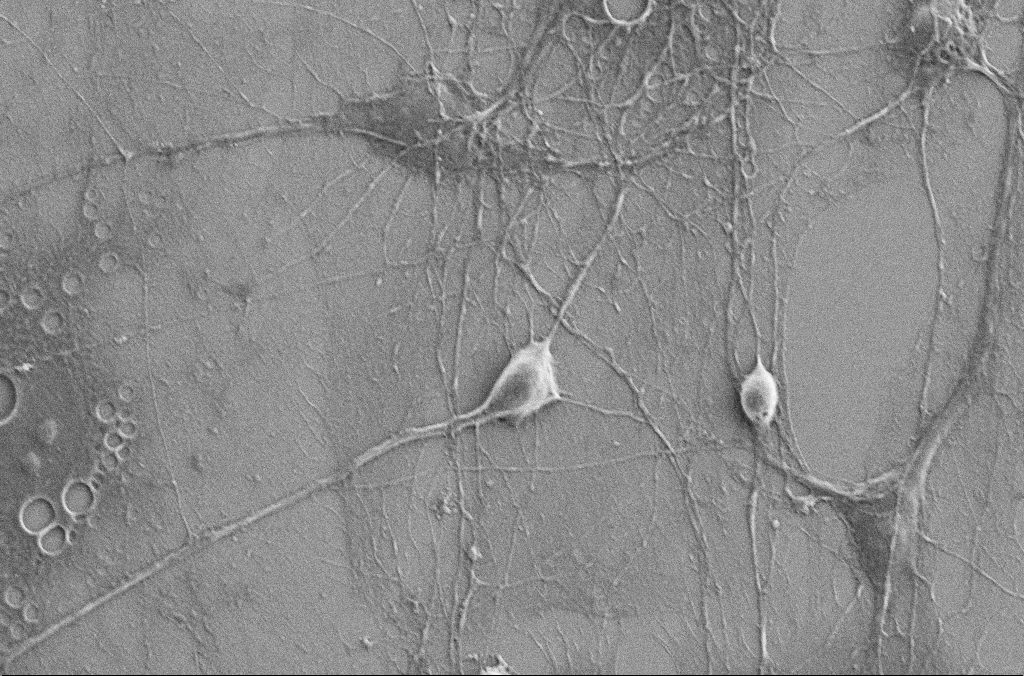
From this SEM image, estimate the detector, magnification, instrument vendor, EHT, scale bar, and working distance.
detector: SE2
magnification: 3 K X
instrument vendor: Zeiss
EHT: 5 kV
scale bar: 10000 nm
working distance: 5.9 mm